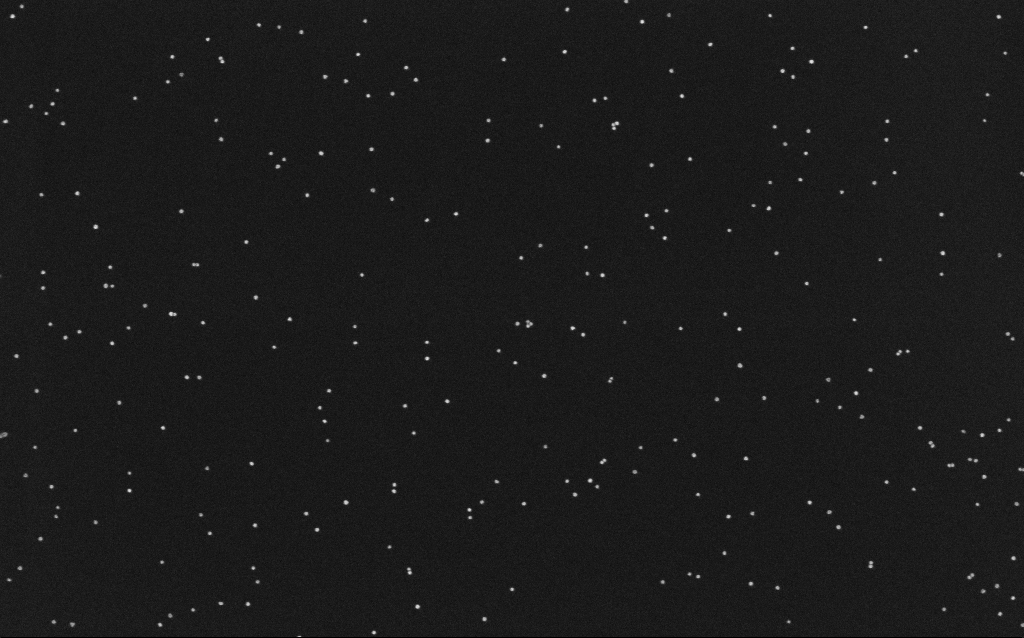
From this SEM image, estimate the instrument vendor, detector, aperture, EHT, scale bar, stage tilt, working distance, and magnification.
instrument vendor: Zeiss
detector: InLens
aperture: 30 µm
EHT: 10 kV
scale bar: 200 nm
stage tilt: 0°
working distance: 6.6 mm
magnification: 100 K X